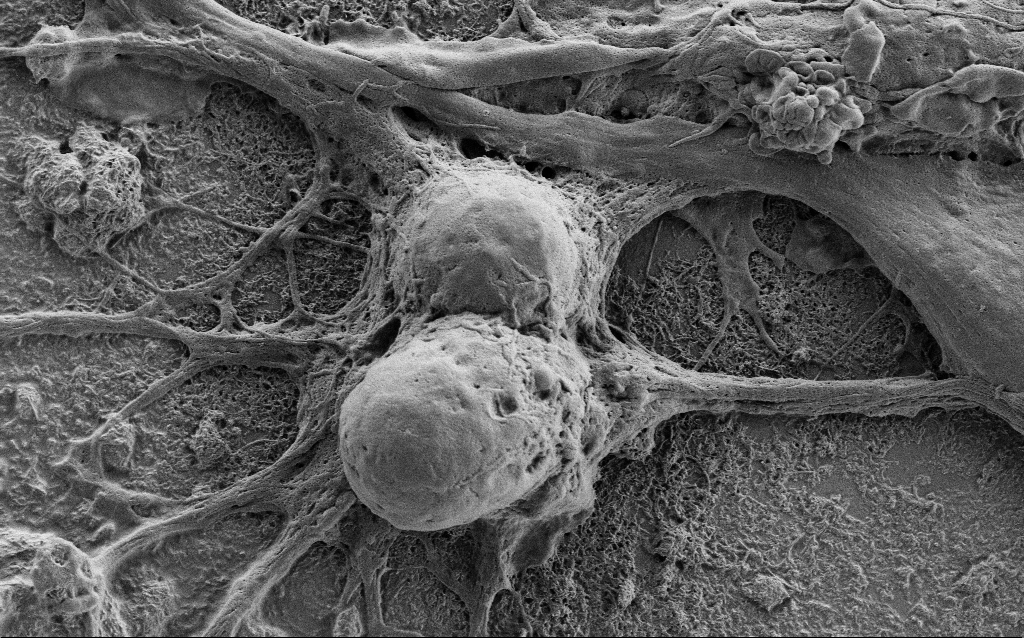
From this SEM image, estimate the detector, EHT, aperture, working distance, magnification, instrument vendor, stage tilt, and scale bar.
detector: SE2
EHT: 1 kV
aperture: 30 µm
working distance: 6 mm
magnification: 10 K X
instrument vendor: Zeiss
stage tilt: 0°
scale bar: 2000 nm